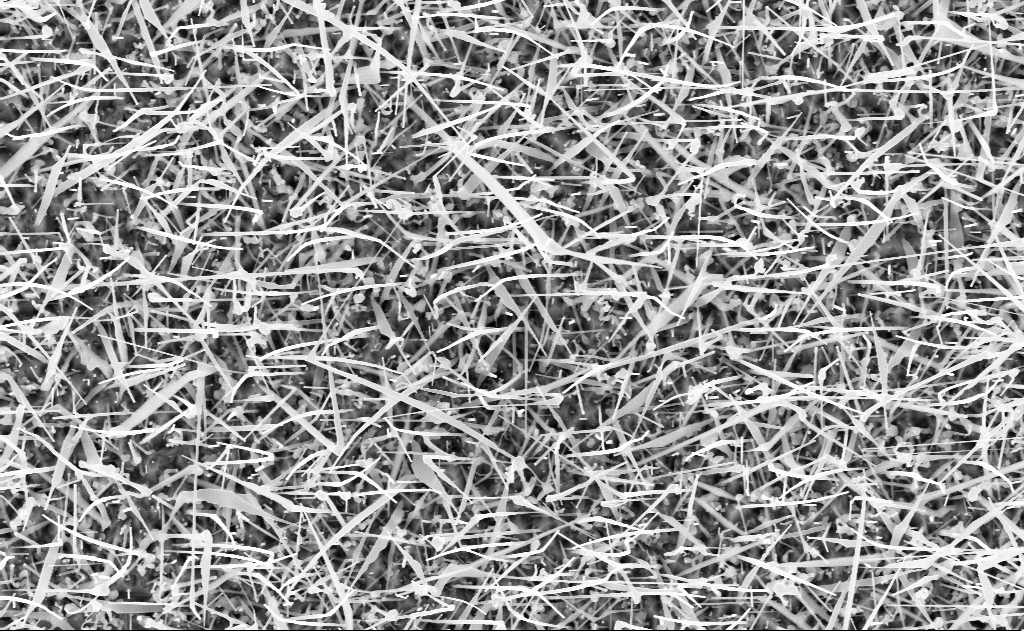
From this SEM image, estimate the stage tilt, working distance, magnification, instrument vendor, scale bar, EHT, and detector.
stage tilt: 0°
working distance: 11 mm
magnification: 20 K X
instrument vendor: Zeiss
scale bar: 1000 nm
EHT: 10 kV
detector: InLens